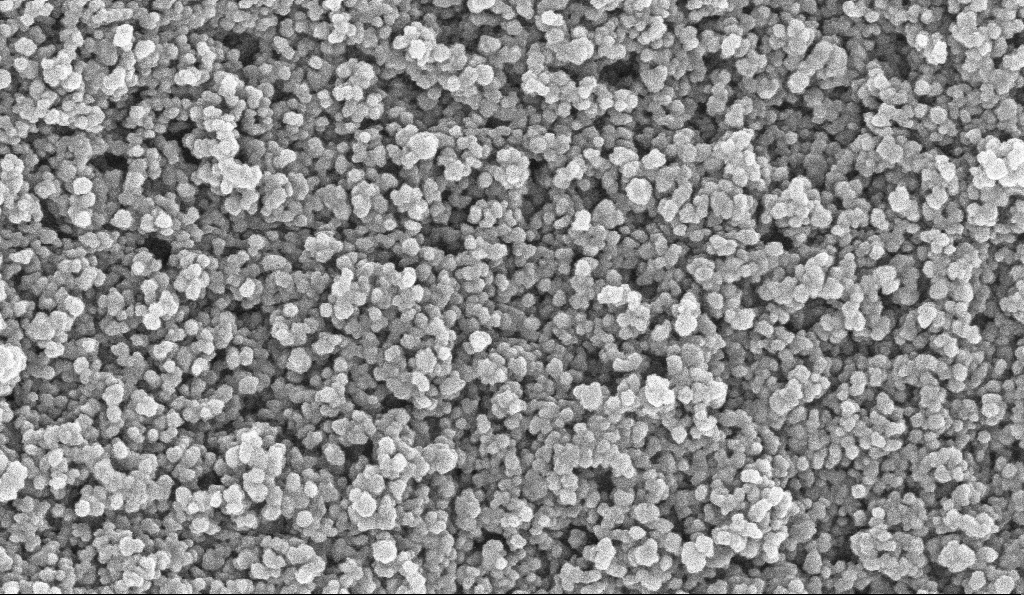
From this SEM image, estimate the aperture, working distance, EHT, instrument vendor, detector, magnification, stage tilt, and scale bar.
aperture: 30 µm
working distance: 6 mm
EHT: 10 kV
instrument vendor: Zeiss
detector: InLens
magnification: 135 K X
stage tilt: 0°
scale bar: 200 nm